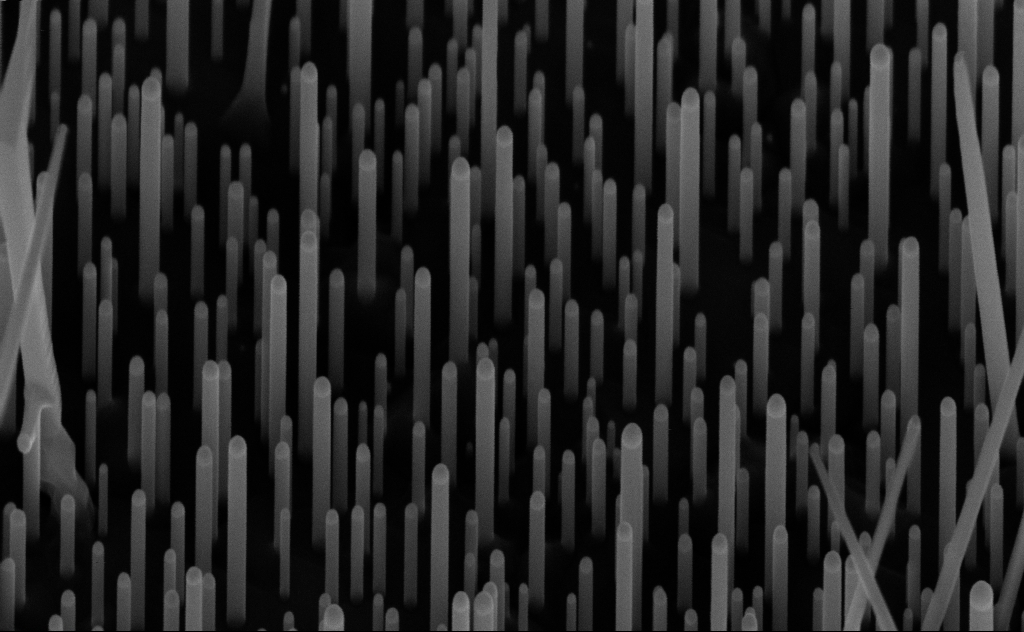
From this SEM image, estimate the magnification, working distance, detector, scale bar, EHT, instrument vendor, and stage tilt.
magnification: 100 K X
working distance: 7 mm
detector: InLens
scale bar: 200 nm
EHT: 10 kV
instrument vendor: Zeiss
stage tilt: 45°